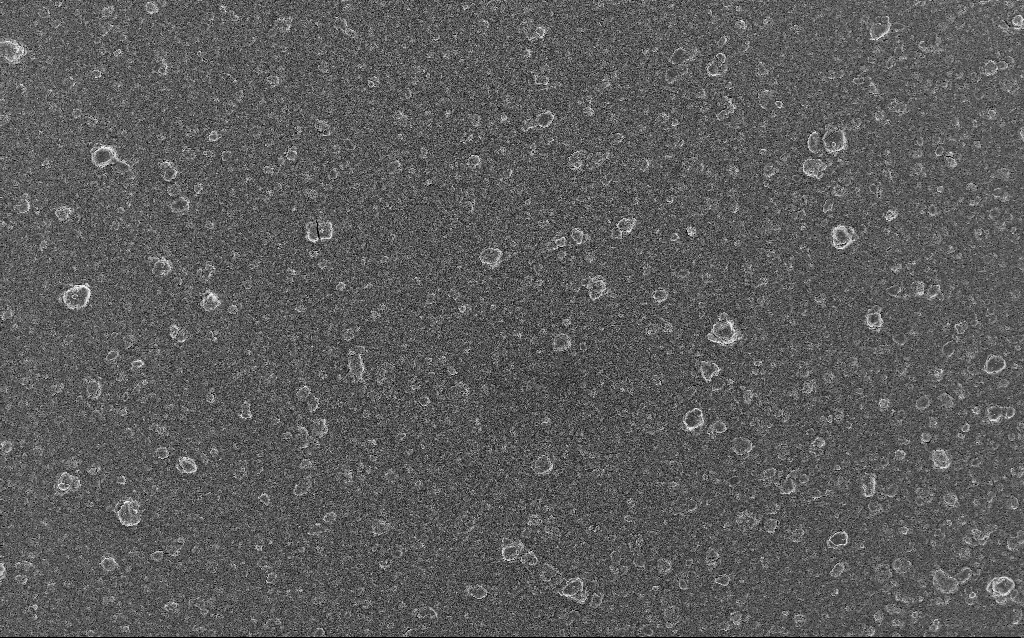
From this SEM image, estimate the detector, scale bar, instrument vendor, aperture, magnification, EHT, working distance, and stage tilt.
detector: InLens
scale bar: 20000 nm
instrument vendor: Zeiss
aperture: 30 µm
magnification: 0.77 K X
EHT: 5 kV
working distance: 5.3 mm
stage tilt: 0°